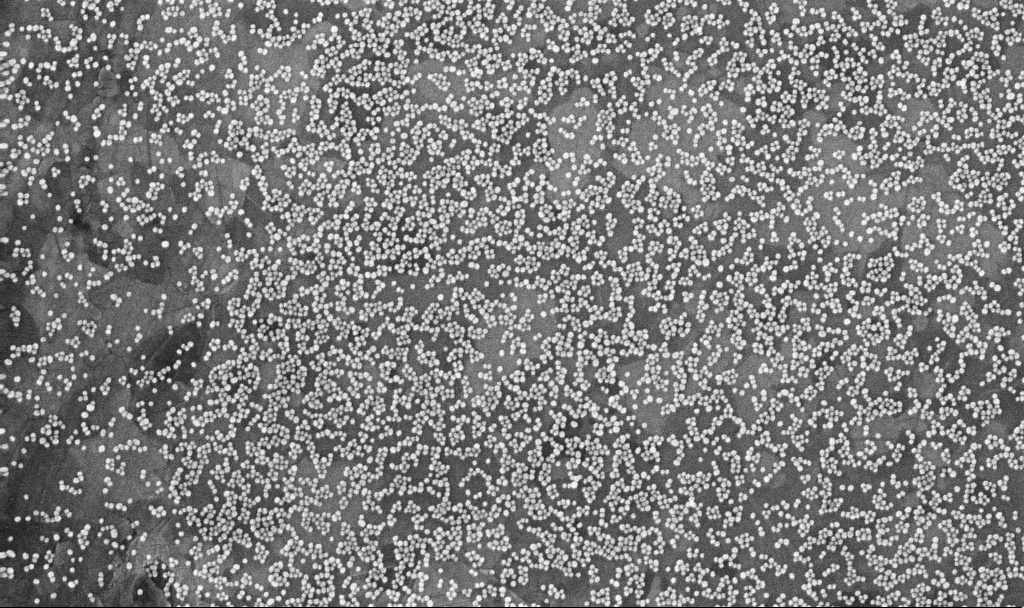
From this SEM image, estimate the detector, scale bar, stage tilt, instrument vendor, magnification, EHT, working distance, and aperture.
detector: InLens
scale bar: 200 nm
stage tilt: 0°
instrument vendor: Zeiss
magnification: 100 K X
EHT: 10 kV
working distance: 3.3 mm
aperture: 30 µm